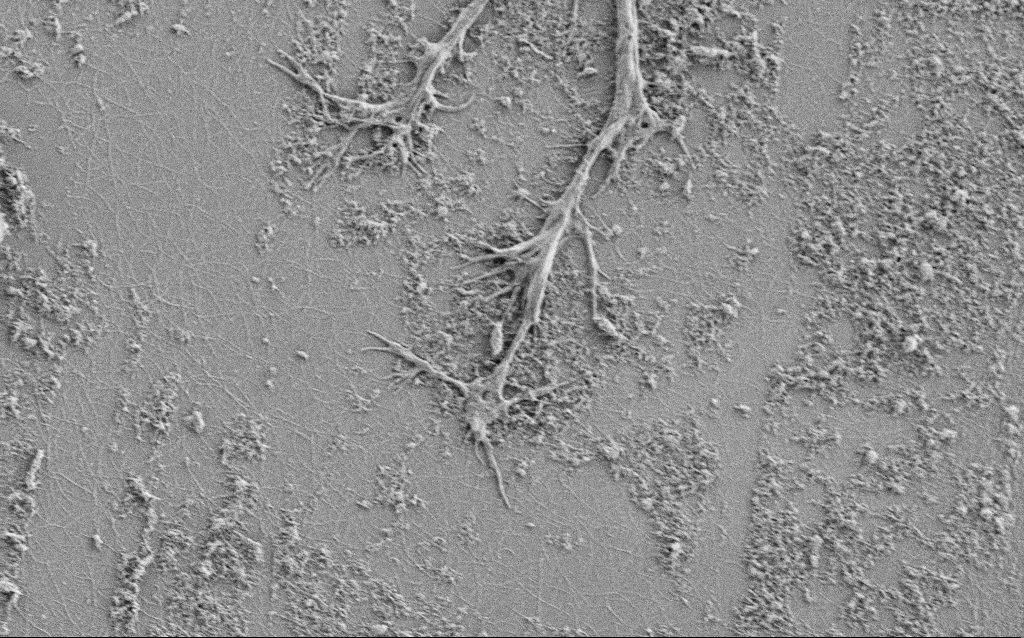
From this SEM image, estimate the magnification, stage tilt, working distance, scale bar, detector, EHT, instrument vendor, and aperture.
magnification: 5 K X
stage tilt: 0°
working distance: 4 mm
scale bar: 10000 nm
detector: SE2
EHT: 1 kV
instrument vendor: Zeiss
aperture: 30 µm